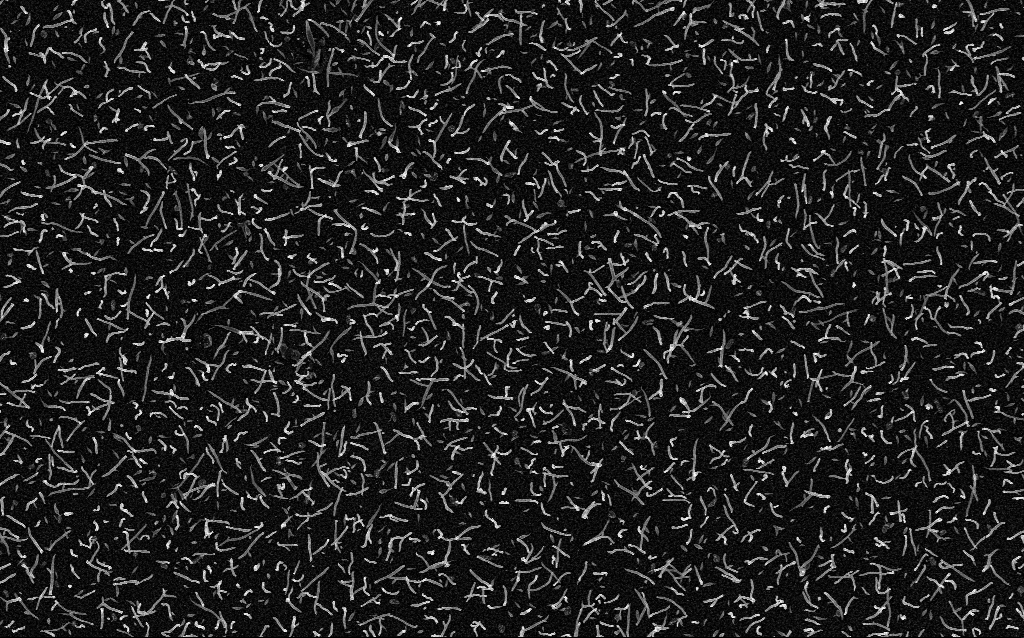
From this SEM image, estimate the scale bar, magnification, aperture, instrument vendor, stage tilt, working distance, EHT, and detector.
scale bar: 2000 nm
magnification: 10 K X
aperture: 30 µm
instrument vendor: Zeiss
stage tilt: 0°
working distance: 1.8 mm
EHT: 5 kV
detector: InLens